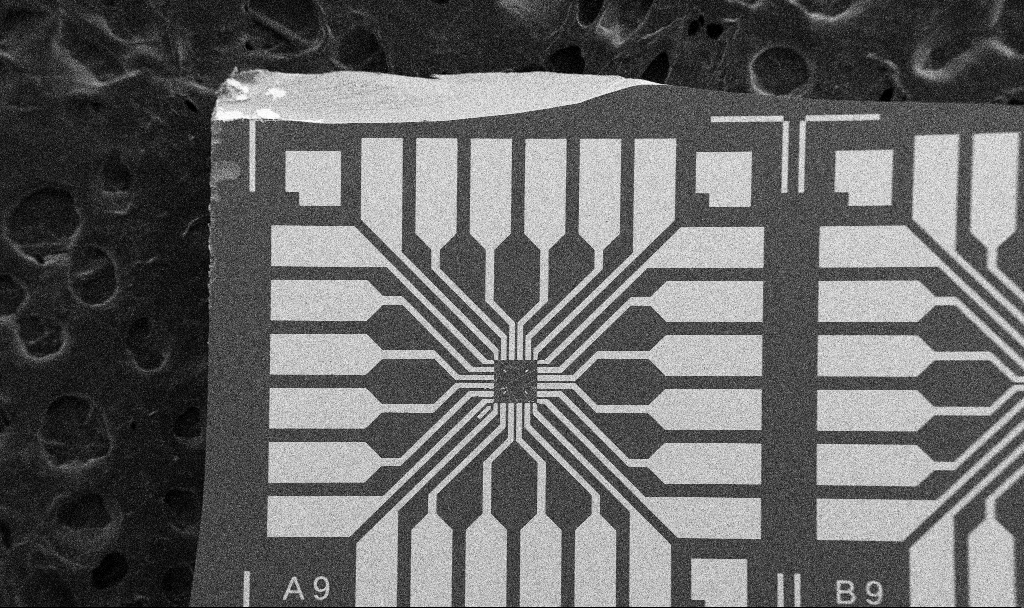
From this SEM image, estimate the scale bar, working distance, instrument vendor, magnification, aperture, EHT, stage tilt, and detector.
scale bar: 200000 nm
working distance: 10.7 mm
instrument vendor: Zeiss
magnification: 0.1 K X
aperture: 30 µm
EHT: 5 kV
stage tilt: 0°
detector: SE2